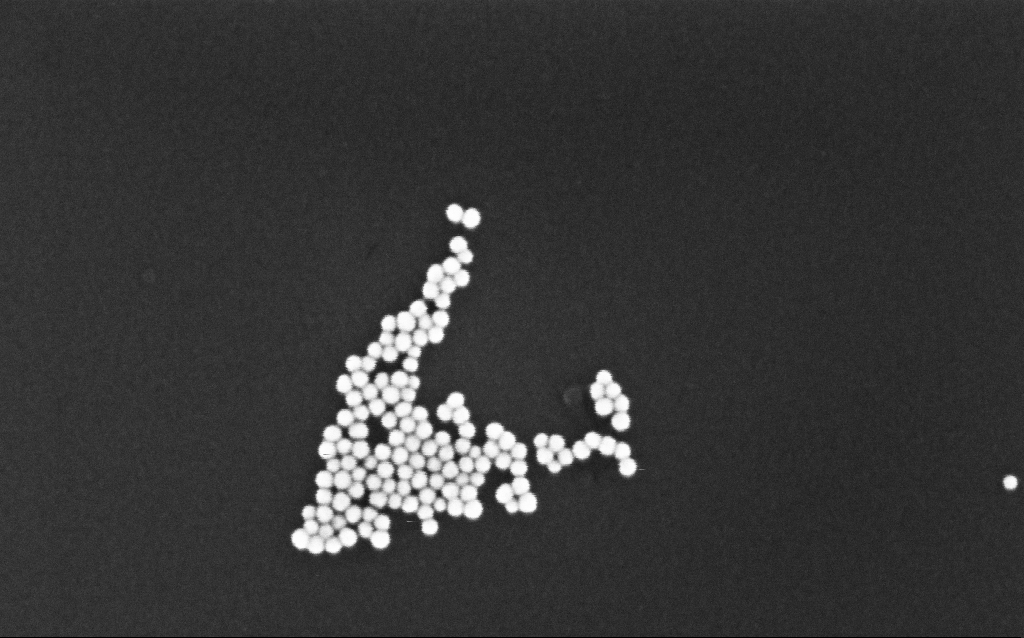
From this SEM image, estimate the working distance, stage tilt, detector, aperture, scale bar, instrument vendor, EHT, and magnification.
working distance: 3.4 mm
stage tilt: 0°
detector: InLens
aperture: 30 µm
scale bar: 100 nm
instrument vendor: Zeiss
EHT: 5 kV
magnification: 300 K X